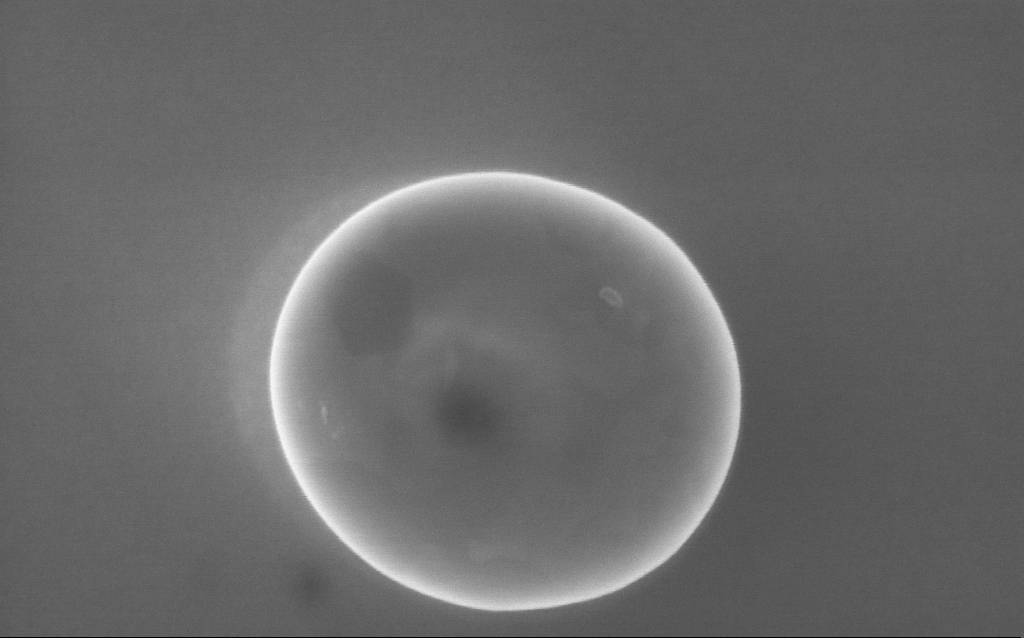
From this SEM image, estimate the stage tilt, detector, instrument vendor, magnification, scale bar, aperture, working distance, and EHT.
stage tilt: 0°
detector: InLens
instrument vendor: Zeiss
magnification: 100 K X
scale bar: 200 nm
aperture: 30 µm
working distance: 3 mm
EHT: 5 kV